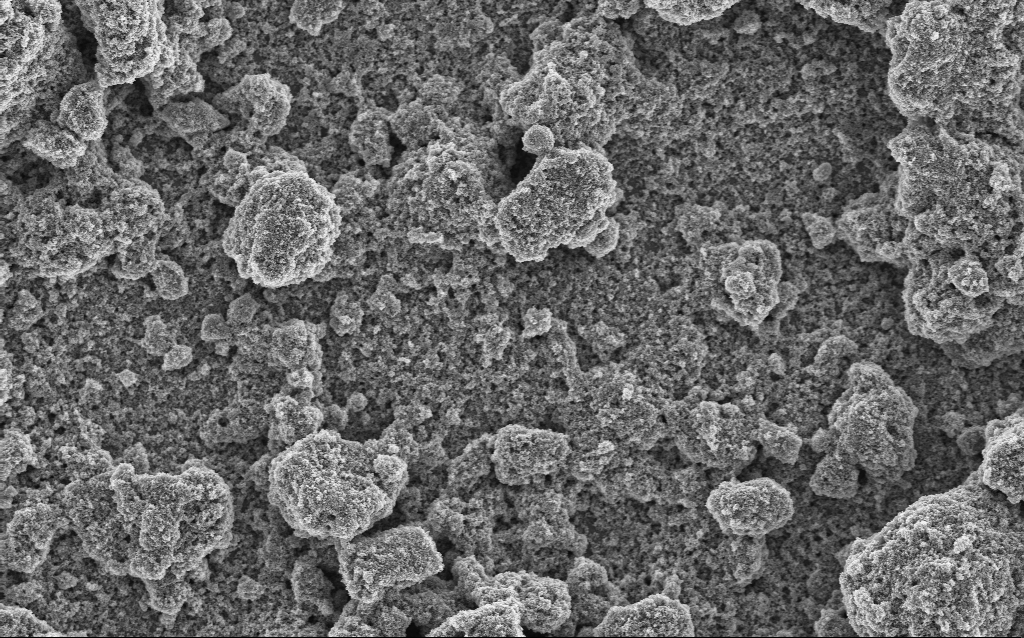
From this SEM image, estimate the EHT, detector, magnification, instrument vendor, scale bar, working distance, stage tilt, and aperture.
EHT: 5 kV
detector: InLens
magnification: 6.41 K X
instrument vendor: Zeiss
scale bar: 10000 nm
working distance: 4.2 mm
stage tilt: -0°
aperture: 30 µm